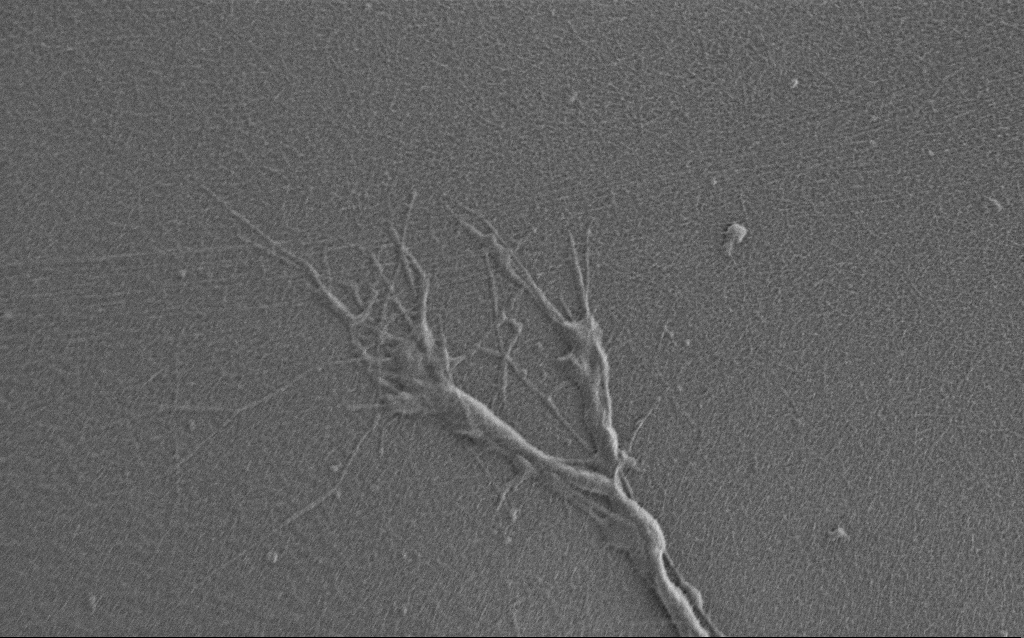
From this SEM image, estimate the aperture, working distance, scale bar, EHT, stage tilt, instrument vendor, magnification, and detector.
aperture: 30 µm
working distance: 7 mm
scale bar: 2000 nm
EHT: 0.9 kV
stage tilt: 0°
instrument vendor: Zeiss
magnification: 7.5 K X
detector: SE2